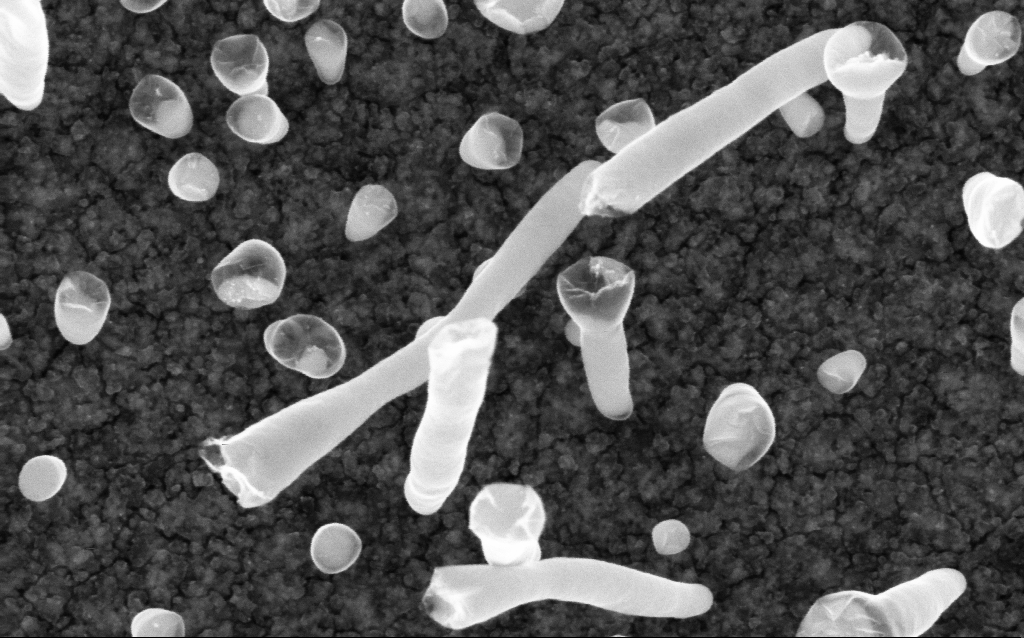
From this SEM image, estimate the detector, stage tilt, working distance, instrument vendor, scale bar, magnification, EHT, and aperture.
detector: InLens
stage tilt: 0°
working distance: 2 mm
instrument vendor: Zeiss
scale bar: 200 nm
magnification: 200 K X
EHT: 5 kV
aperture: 30 µm